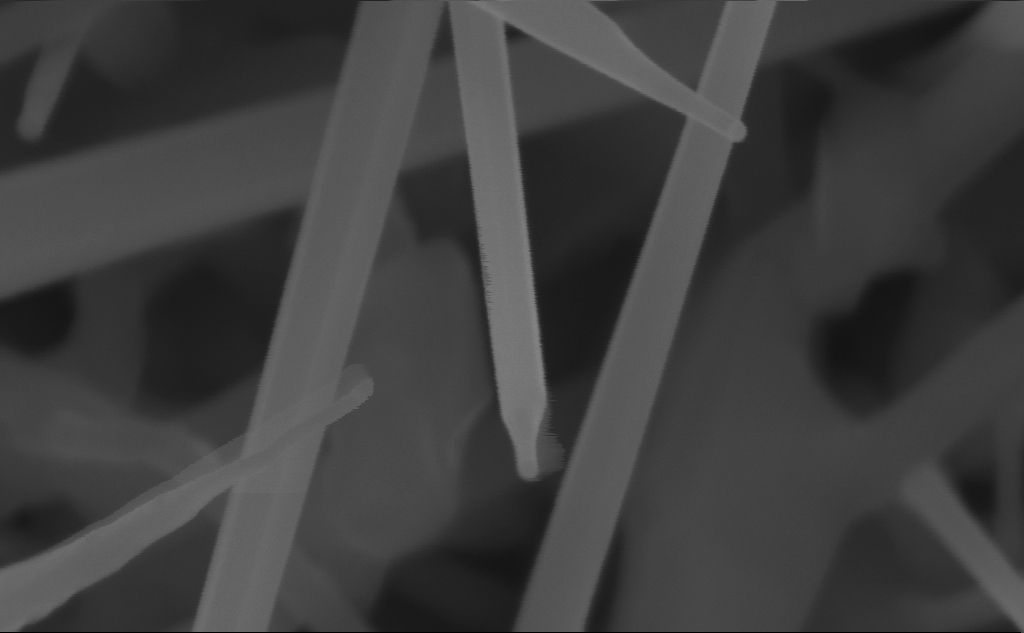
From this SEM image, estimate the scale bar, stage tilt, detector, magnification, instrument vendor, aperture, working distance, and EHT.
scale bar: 100 nm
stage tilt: -0°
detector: InLens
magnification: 318.89 K X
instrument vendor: Zeiss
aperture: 30 µm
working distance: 7 mm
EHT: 10 kV